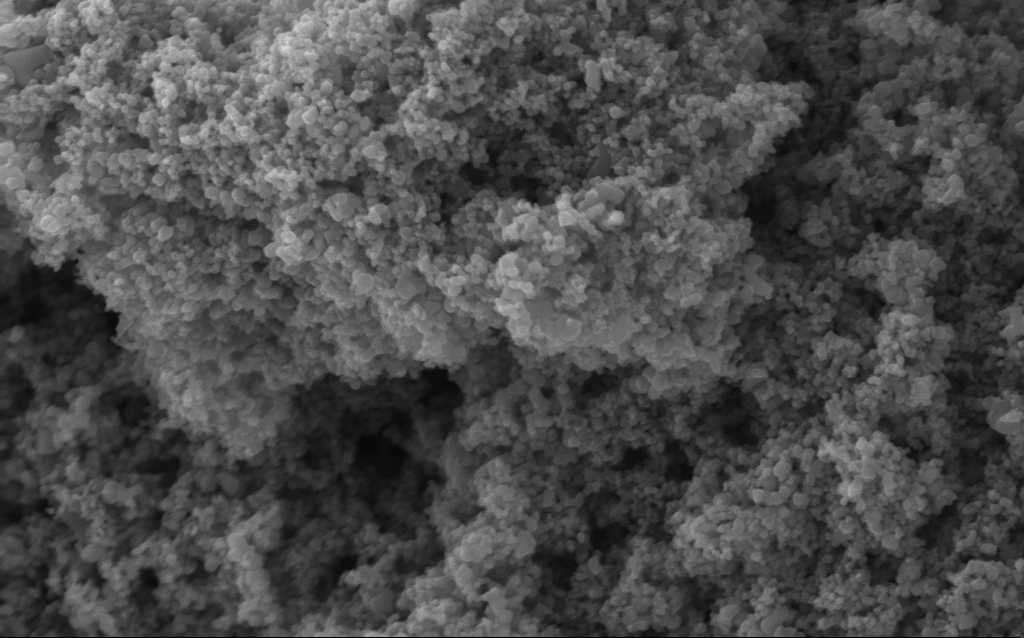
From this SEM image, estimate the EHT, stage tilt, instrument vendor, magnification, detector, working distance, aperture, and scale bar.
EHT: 5 kV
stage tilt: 0°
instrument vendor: Zeiss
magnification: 114.56 K X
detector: InLens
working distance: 4.2 mm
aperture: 30 µm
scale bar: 200 nm